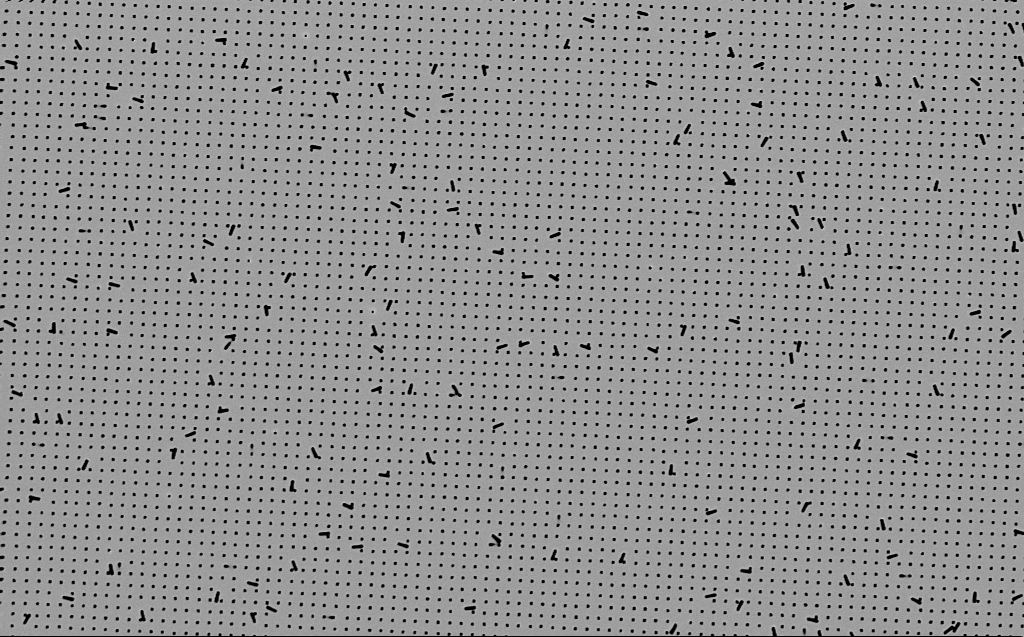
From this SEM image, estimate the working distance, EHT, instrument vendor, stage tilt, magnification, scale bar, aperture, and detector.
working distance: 6 mm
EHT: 3 kV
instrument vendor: Zeiss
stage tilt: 0°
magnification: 8.5 K X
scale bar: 2000 nm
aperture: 30 µm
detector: InLens